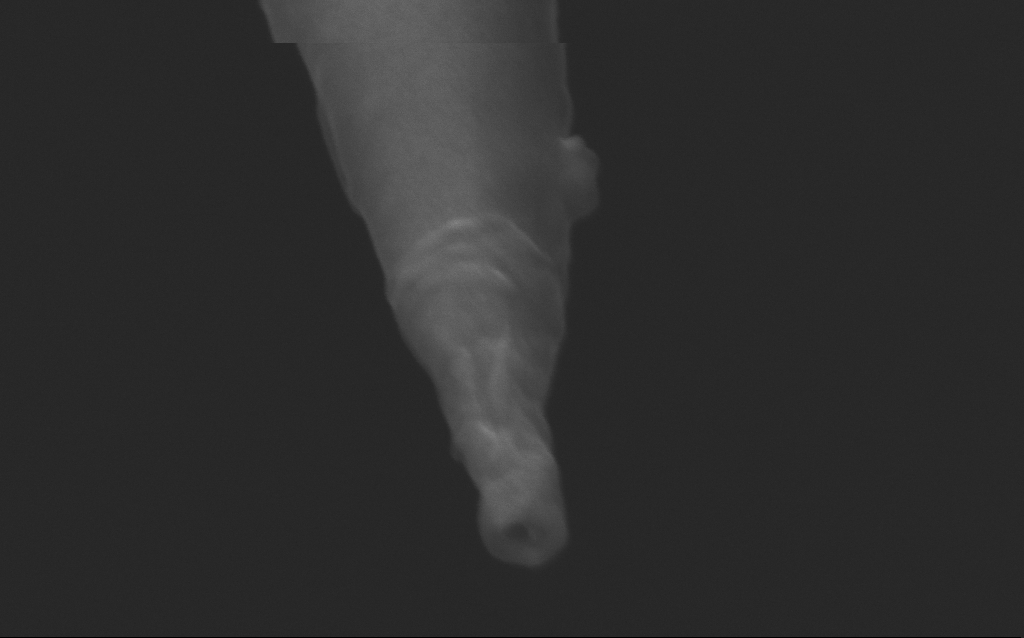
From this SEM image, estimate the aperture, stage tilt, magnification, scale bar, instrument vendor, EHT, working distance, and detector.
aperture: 30 µm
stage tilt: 45°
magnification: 287.17 K X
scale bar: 200 nm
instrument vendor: Zeiss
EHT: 5 kV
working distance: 6 mm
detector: InLens